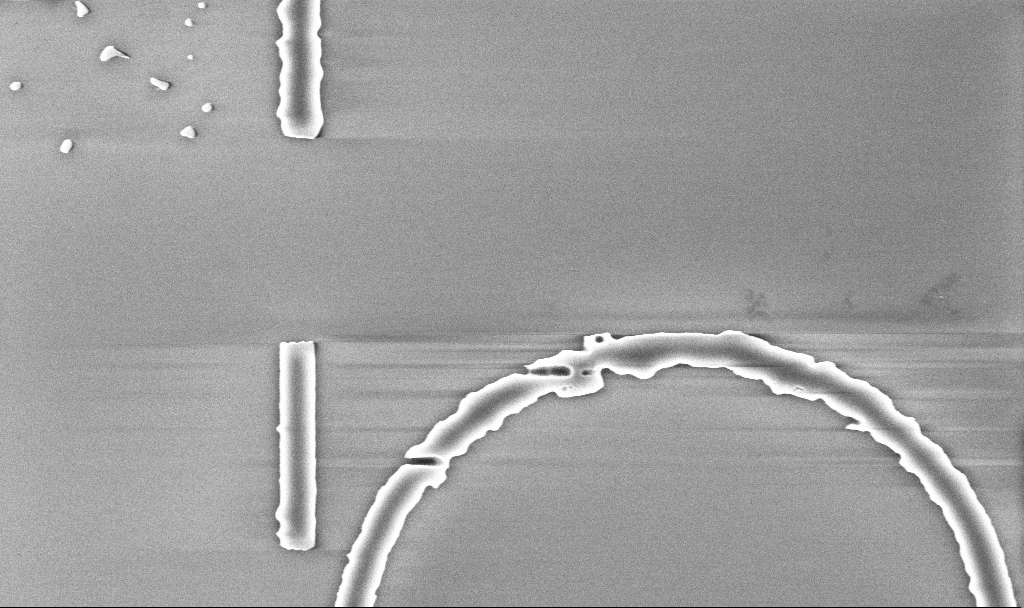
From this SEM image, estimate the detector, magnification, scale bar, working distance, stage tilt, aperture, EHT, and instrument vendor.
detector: InLens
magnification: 25.8 K X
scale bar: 2000 nm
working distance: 5.2 mm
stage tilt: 0°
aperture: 30 µm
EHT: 5 kV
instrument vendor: Zeiss